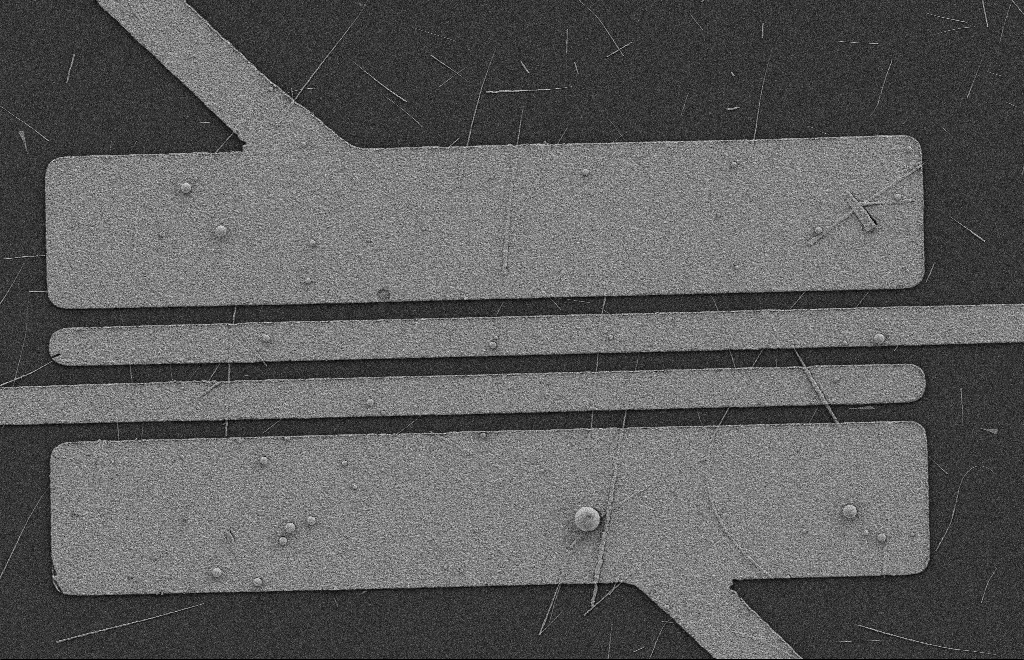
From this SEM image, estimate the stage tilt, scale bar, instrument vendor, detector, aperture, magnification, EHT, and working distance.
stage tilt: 0°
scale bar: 2000 nm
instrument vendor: Zeiss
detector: SE2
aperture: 20 µm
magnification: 5.26 K X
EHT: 2 kV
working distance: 9 mm